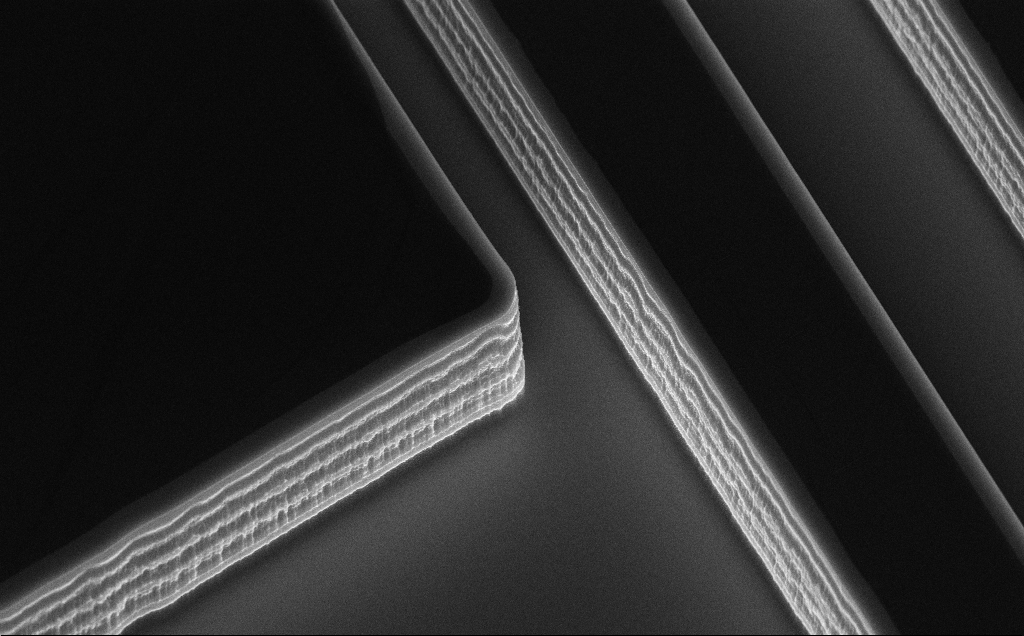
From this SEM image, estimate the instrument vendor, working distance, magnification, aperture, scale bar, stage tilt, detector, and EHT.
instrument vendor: Zeiss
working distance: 11 mm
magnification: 7.3 K X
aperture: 30 µm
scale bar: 2000 nm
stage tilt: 50°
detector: InLens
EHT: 10 kV